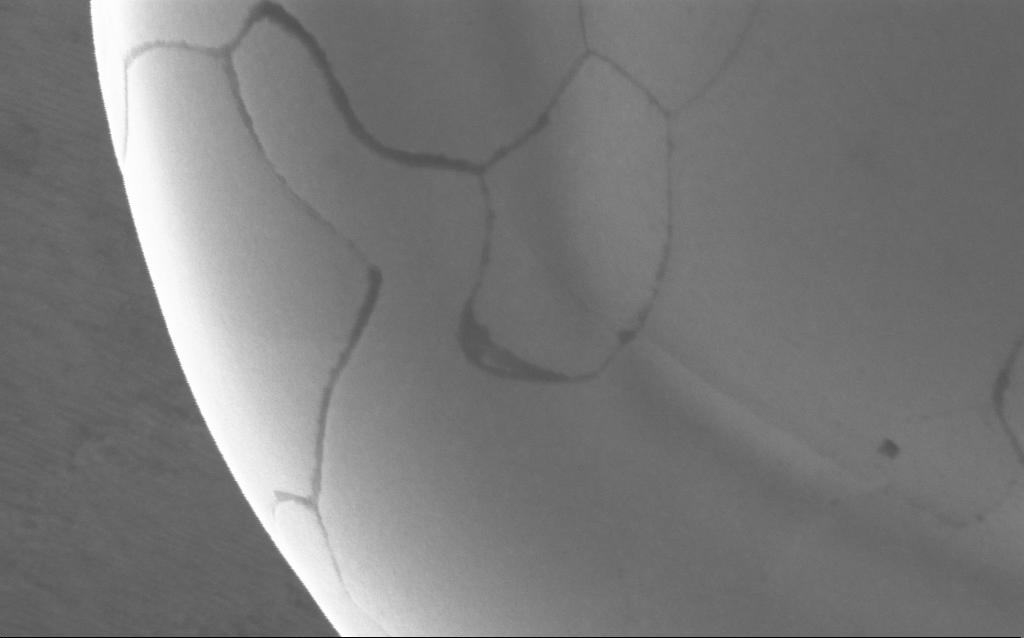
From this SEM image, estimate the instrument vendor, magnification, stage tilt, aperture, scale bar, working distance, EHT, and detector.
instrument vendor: Zeiss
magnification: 194.95 K X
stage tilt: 0°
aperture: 30 µm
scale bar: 200 nm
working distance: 3 mm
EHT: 5 kV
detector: InLens